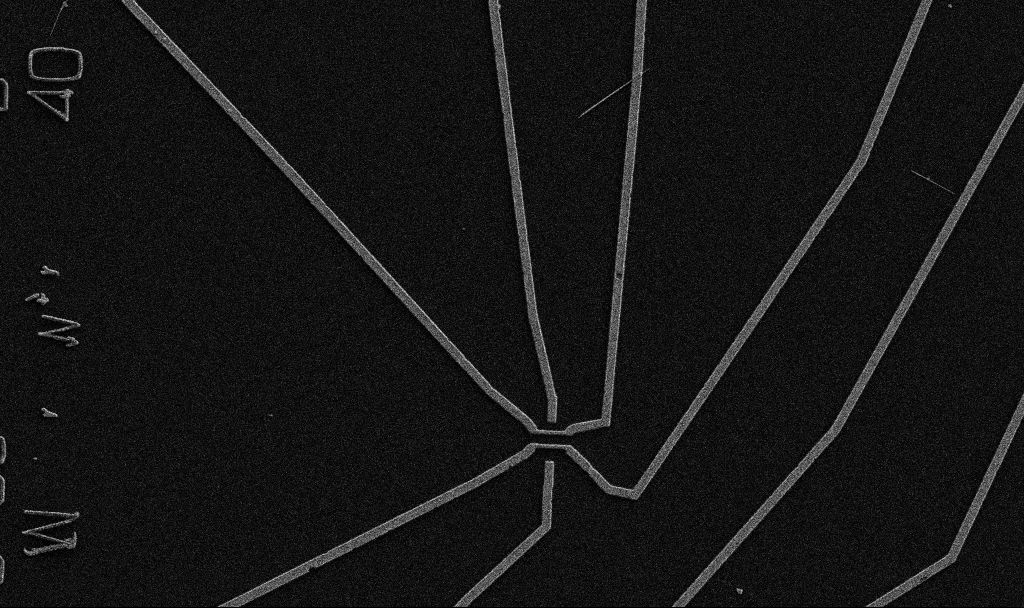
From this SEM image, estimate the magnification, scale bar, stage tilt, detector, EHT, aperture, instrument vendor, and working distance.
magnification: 5 K X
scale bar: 10000 nm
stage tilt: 0°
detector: SE2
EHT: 5 kV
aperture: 30 µm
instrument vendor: Zeiss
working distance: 10.7 mm